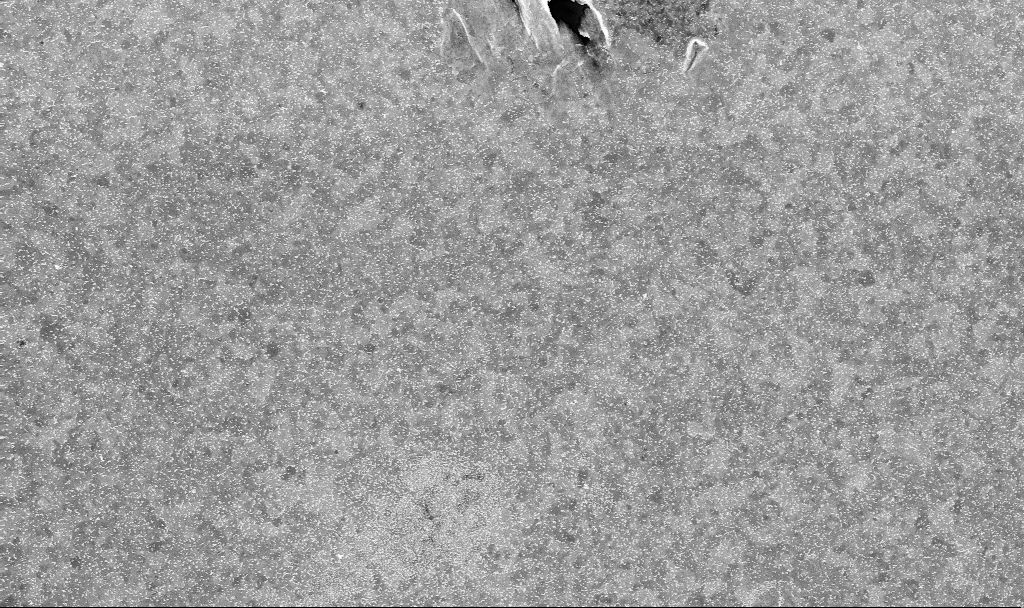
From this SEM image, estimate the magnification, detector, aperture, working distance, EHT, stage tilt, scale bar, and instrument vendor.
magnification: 20 K X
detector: InLens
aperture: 30 µm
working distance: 3.8 mm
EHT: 10 kV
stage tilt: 0°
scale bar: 1000 nm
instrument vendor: Zeiss